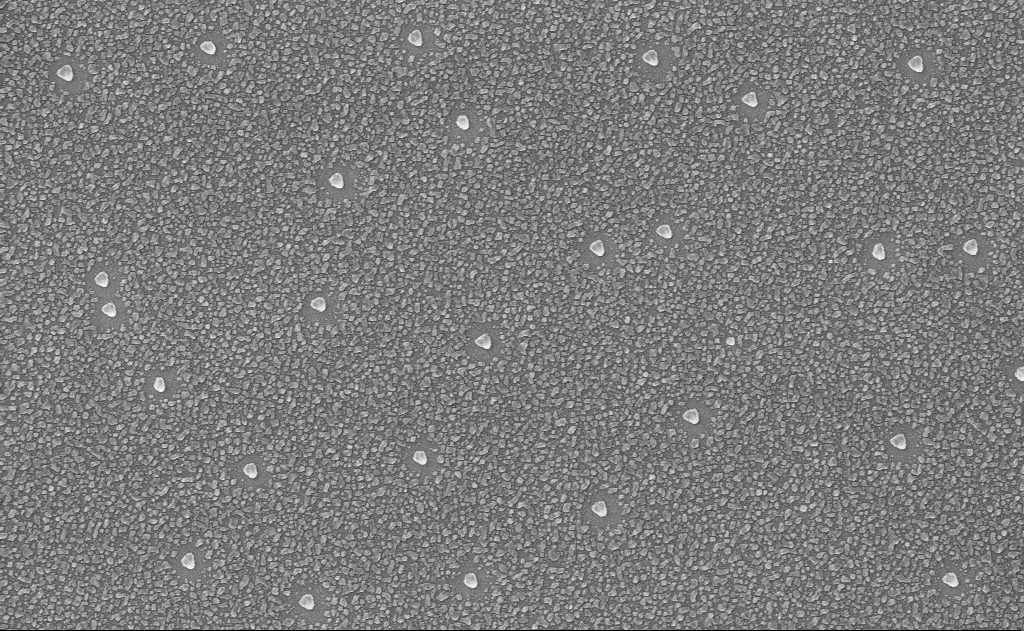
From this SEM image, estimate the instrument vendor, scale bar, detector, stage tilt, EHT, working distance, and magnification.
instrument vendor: Zeiss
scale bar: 1000 nm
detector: InLens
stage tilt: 0°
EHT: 10 kV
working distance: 15 mm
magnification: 40 K X